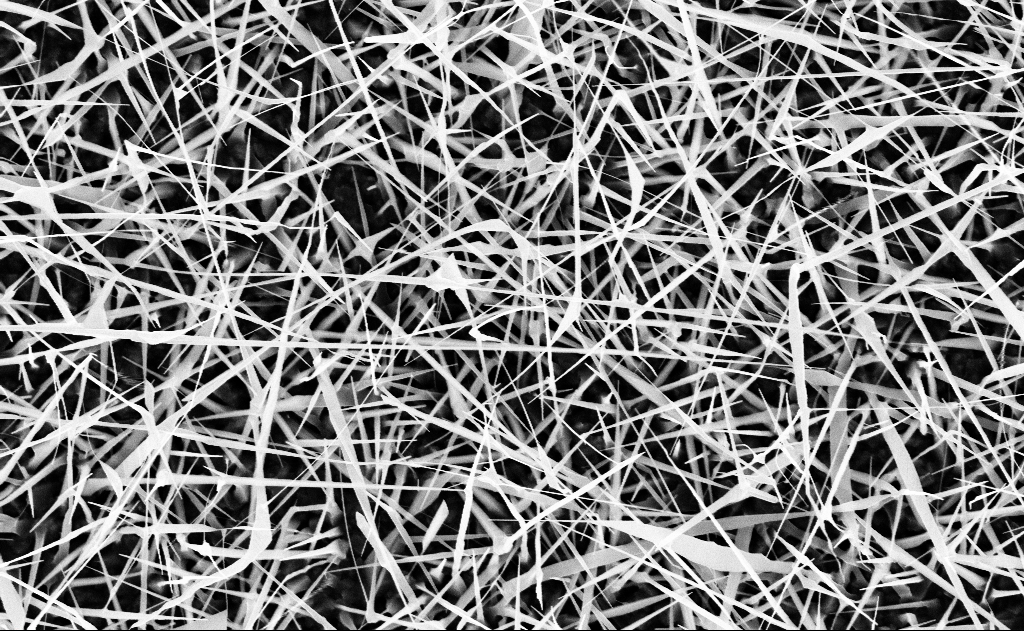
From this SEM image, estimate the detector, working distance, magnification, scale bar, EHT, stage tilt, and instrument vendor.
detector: InLens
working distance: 15 mm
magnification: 20 K X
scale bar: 2000 nm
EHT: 10 kV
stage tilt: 0°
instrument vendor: Zeiss